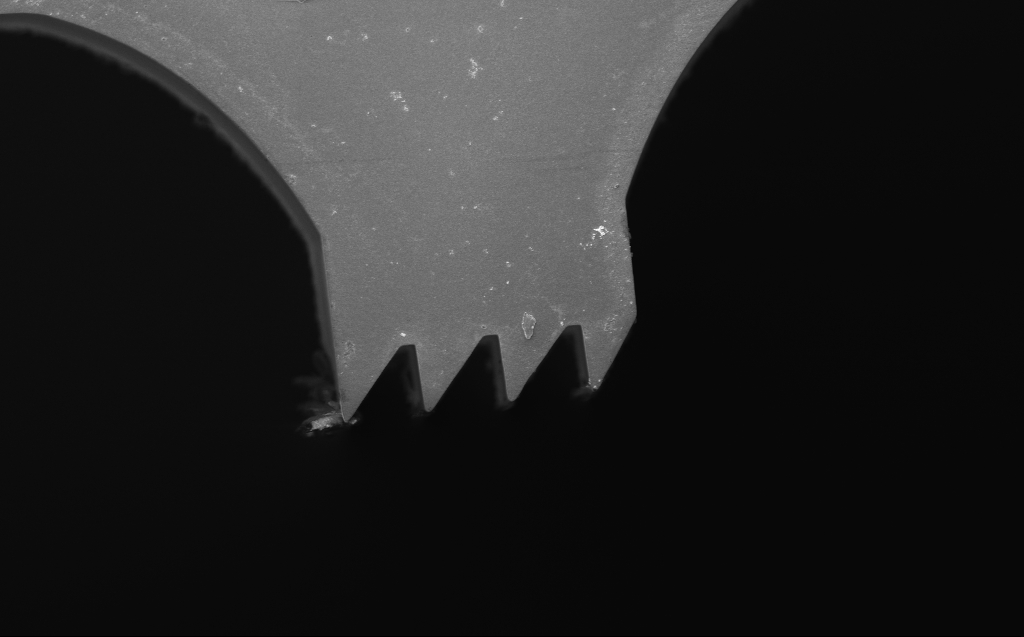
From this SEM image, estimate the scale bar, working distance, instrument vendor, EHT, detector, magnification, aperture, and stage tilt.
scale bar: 10000 nm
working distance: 5 mm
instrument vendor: Zeiss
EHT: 3 kV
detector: InLens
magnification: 2.71 K X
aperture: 30 µm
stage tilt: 0°